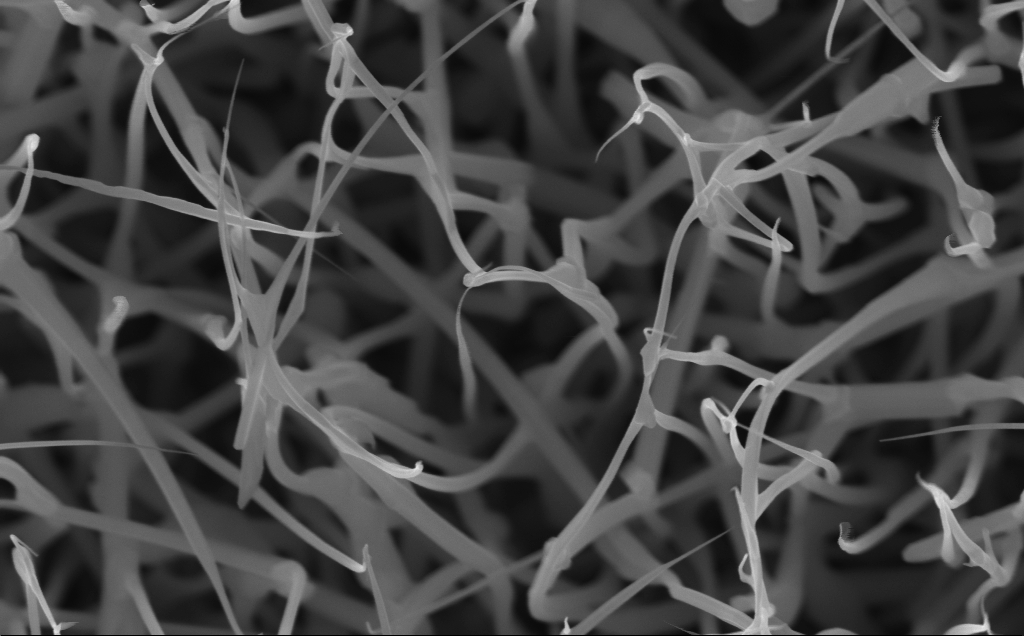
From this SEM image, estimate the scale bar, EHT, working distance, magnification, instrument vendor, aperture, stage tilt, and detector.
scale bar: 200 nm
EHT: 10 kV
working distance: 5 mm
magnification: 80 K X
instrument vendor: Zeiss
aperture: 30 µm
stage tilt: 0°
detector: InLens